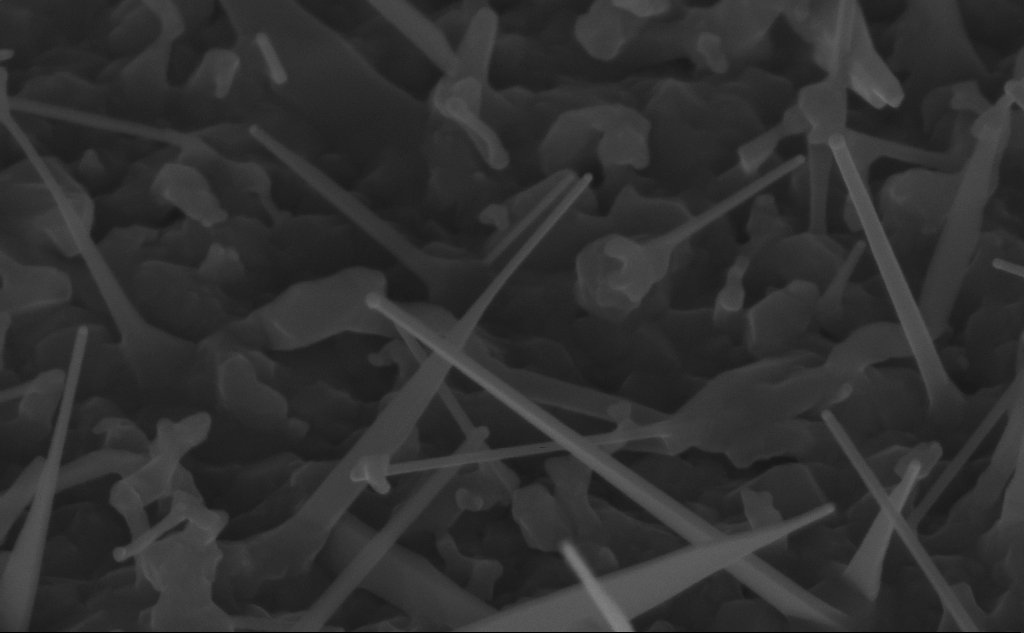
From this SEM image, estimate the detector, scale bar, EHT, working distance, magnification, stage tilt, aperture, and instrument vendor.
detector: InLens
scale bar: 100 nm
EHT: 10 kV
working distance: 8 mm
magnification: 150 K X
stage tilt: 45°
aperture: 30 µm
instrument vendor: Zeiss